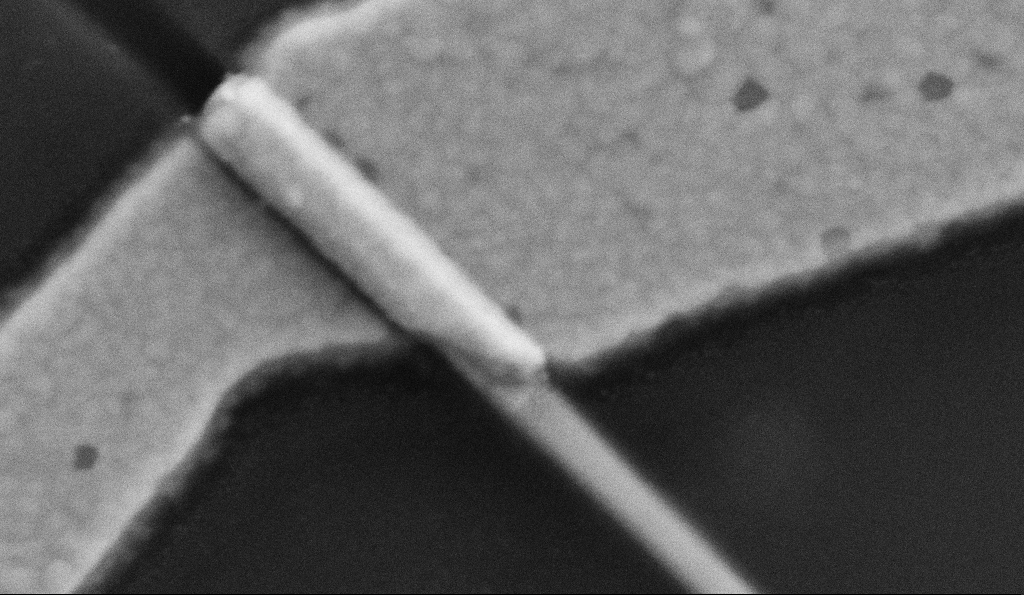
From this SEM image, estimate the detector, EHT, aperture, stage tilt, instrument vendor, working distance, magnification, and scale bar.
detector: SE2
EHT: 5 kV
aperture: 30 µm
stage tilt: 0°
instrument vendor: Zeiss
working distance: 7.7 mm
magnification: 200 K X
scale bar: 200 nm